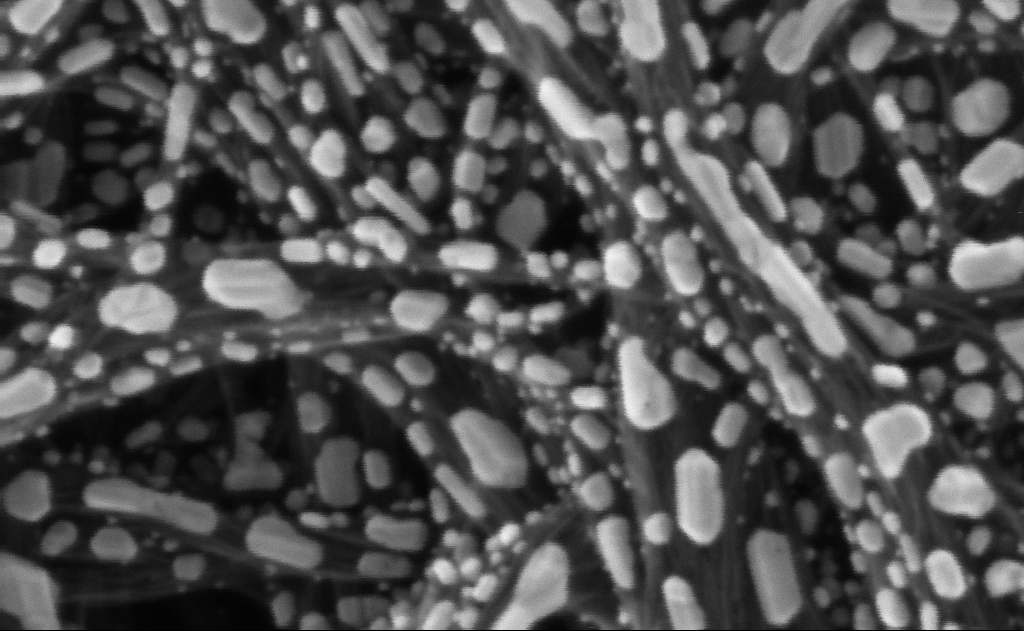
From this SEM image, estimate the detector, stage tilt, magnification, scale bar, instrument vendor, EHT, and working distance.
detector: InLens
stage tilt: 0°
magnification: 834.07 K X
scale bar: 20 nm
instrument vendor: Zeiss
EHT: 10 kV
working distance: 3 mm